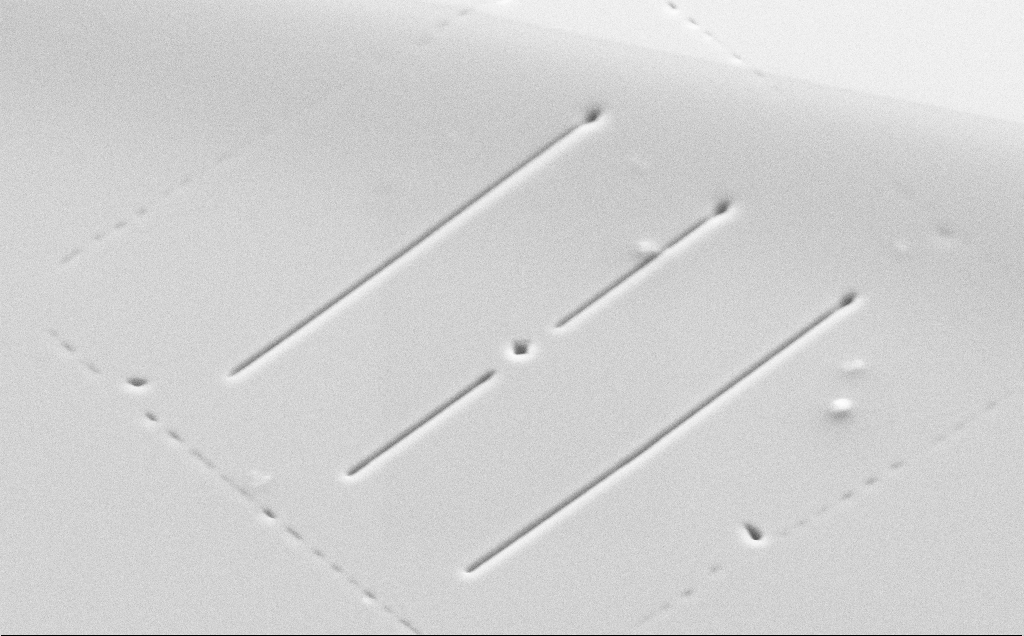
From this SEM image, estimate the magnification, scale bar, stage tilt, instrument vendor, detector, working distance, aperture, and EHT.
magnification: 3.35 K X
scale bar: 20000 nm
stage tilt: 45°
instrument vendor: Zeiss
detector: SE2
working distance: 13 mm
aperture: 30 µm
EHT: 10 kV